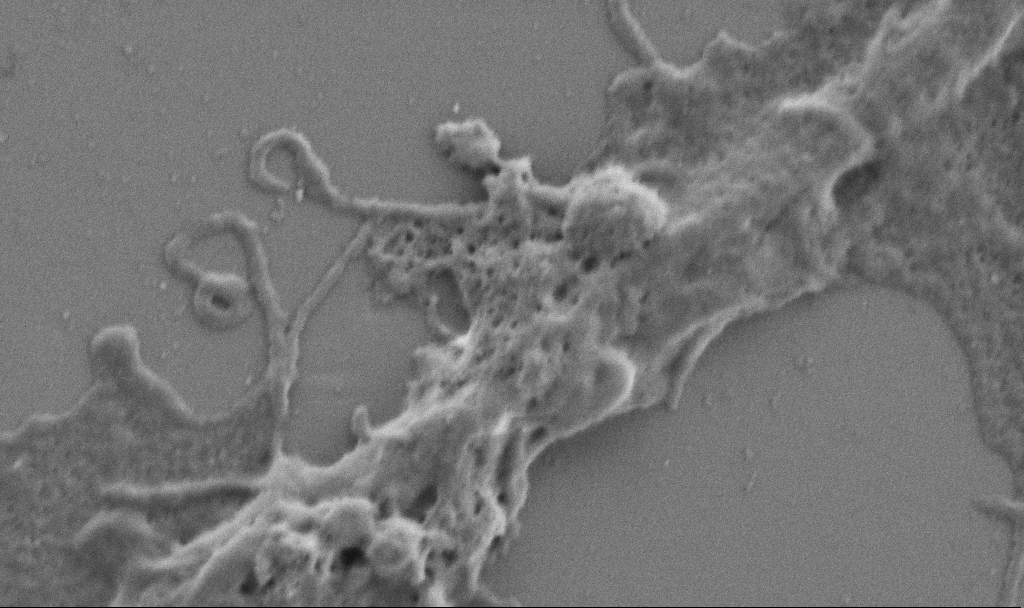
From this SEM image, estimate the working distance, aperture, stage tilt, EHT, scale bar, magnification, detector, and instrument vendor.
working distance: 6.9 mm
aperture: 30 µm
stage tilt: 0°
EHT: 1 kV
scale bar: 1000 nm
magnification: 50 K X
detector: SE2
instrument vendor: Zeiss